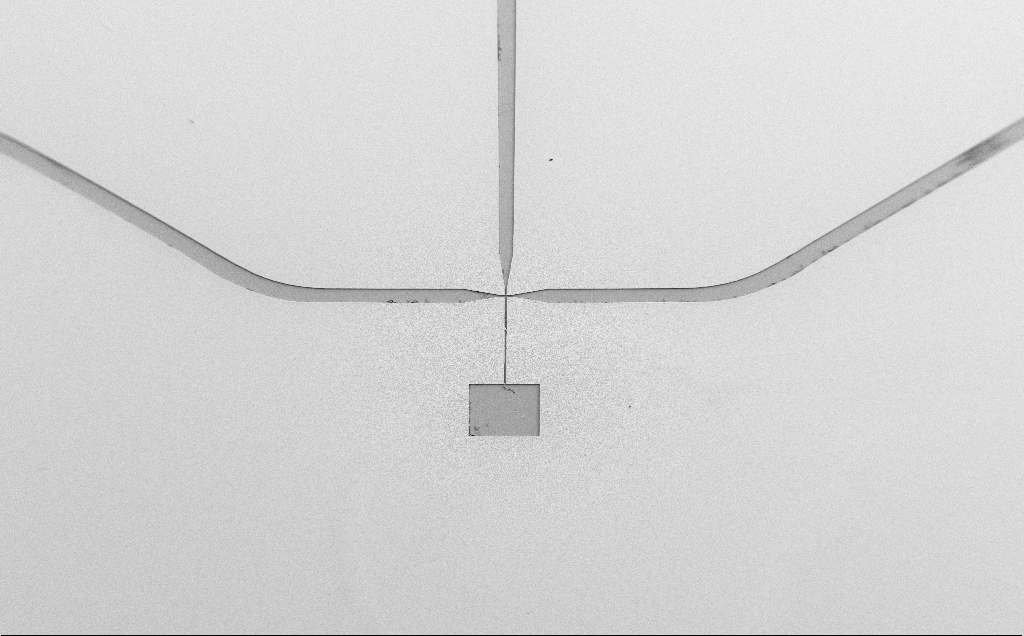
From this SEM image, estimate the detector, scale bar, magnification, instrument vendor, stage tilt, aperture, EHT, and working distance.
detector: SE2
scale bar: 1e+06 nm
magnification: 0.066 K X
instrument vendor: Zeiss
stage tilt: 0°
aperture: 30 µm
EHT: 5 kV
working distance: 13 mm